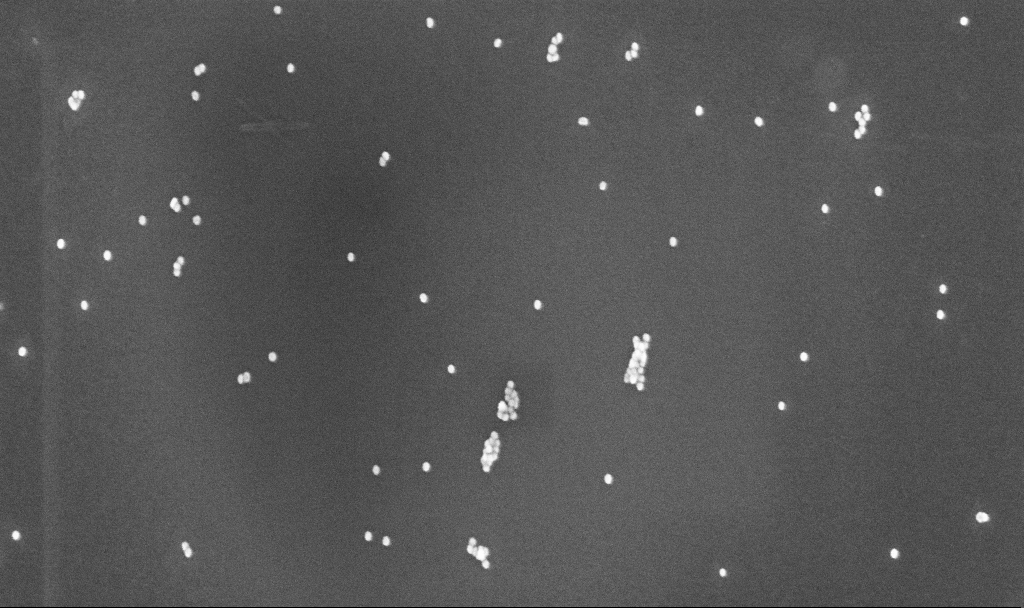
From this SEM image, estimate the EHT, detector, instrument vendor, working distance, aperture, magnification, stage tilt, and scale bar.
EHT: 10 kV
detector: InLens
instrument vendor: Zeiss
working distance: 4.8 mm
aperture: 30 µm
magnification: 100 K X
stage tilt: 0°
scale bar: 200 nm